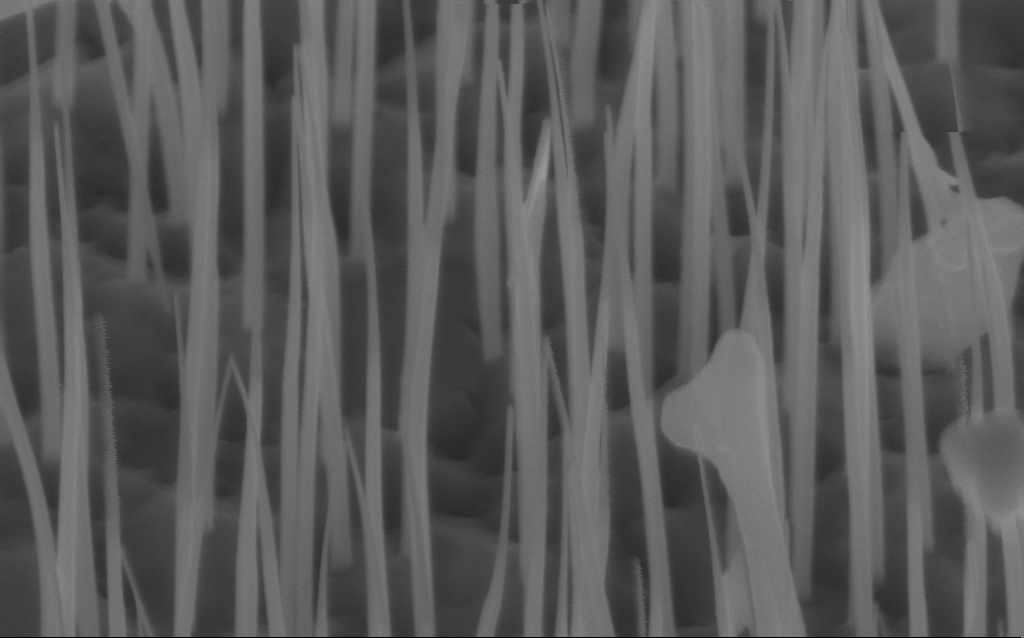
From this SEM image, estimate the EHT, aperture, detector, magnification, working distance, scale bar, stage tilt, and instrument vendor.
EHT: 10 kV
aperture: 30 µm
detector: InLens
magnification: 102.81 K X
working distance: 6 mm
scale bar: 200 nm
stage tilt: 45°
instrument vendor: Zeiss